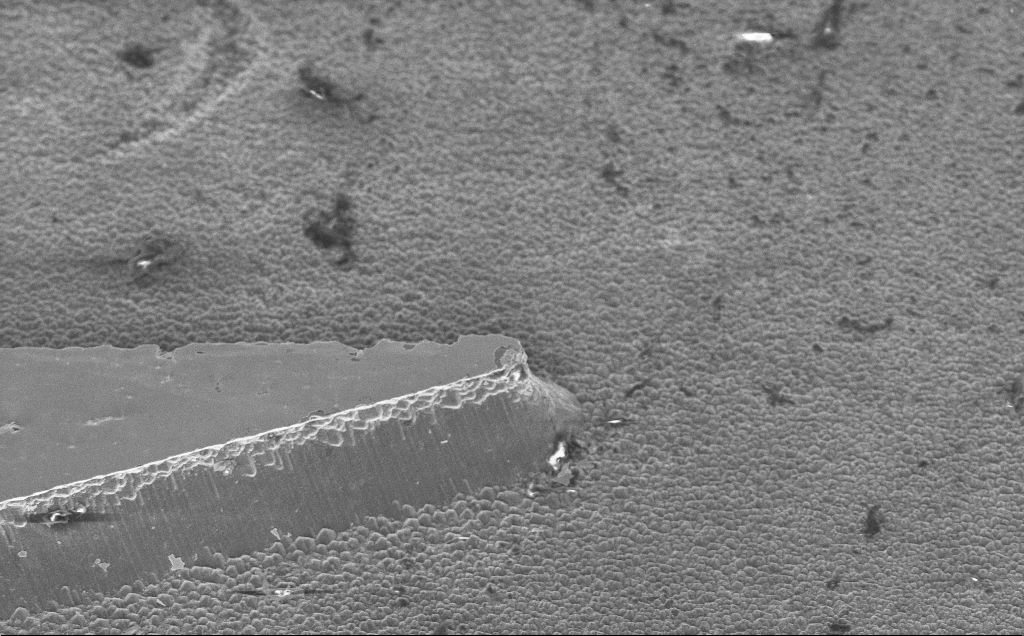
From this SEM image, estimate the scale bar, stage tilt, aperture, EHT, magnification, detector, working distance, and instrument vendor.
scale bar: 20000 nm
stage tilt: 45°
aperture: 30 µm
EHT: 5 kV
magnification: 2.62 K X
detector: InLens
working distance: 13 mm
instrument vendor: Zeiss